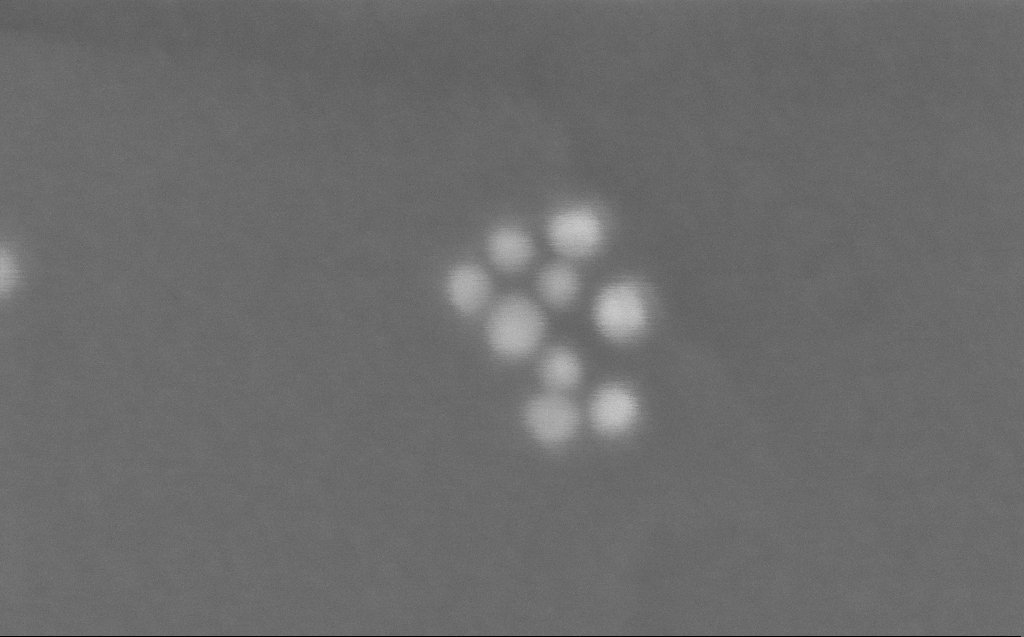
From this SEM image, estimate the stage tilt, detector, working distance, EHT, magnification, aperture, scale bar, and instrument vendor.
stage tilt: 0°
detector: InLens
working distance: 3 mm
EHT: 10 kV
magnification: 1159.8 K X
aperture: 30 µm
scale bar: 20 nm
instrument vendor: Zeiss